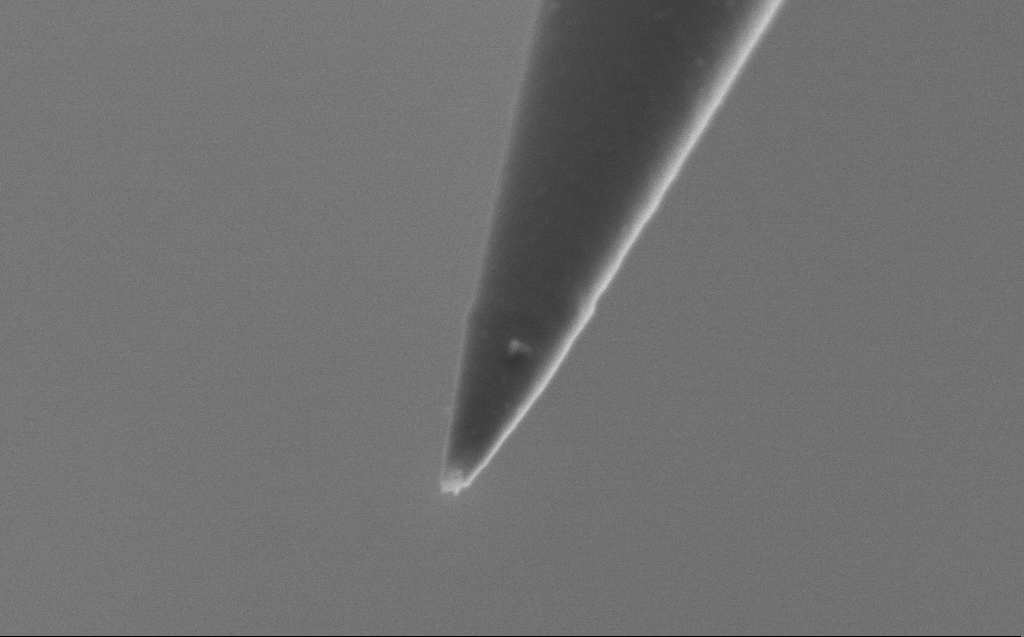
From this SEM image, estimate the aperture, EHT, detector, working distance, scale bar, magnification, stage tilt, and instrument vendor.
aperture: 30 µm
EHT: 2 kV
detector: SE2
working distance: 4 mm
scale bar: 200 nm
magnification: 100 K X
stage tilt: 45°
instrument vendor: Zeiss